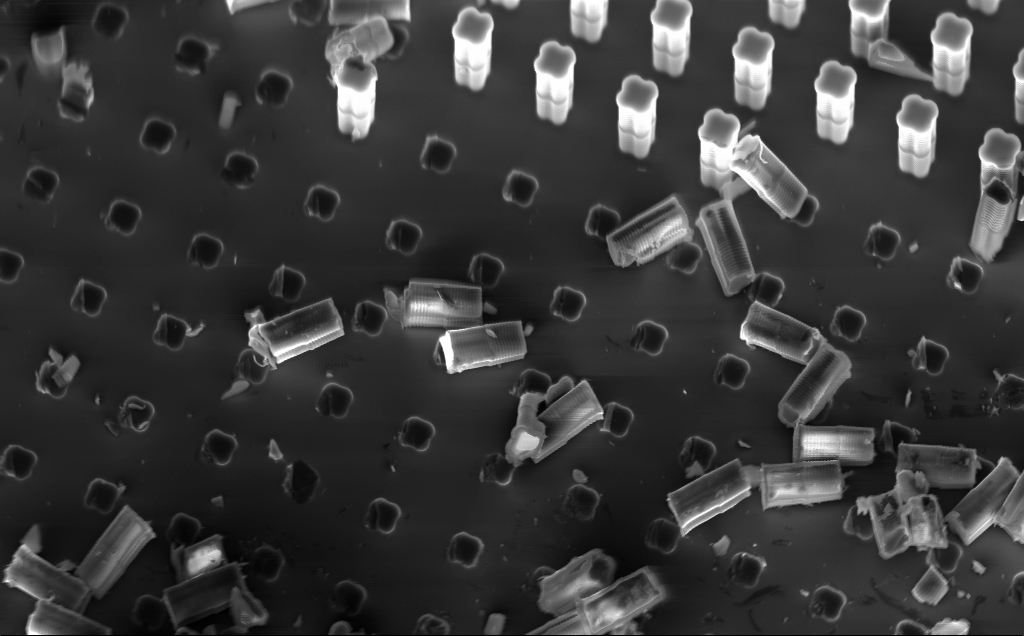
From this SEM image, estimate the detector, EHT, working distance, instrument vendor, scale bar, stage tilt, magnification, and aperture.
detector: InLens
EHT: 10 kV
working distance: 9 mm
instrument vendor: Zeiss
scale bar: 10000 nm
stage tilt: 30.3°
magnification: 3.27 K X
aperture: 120 µm